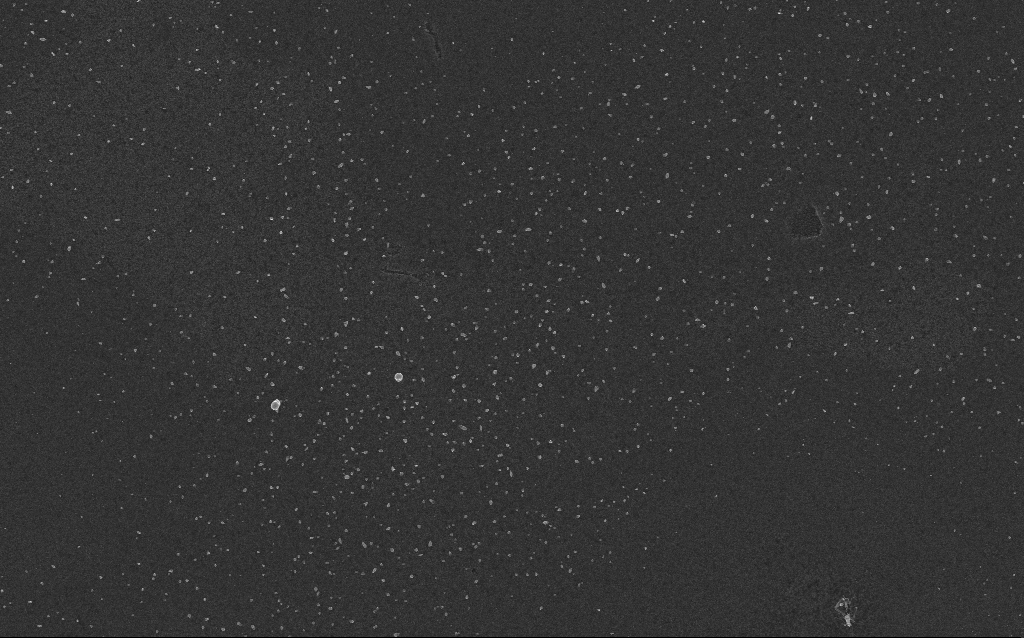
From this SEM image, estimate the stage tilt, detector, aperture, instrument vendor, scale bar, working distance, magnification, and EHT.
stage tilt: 0°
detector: InLens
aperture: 30 µm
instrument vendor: Zeiss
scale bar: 2000 nm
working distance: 2.6 mm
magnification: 10 K X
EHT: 5 kV